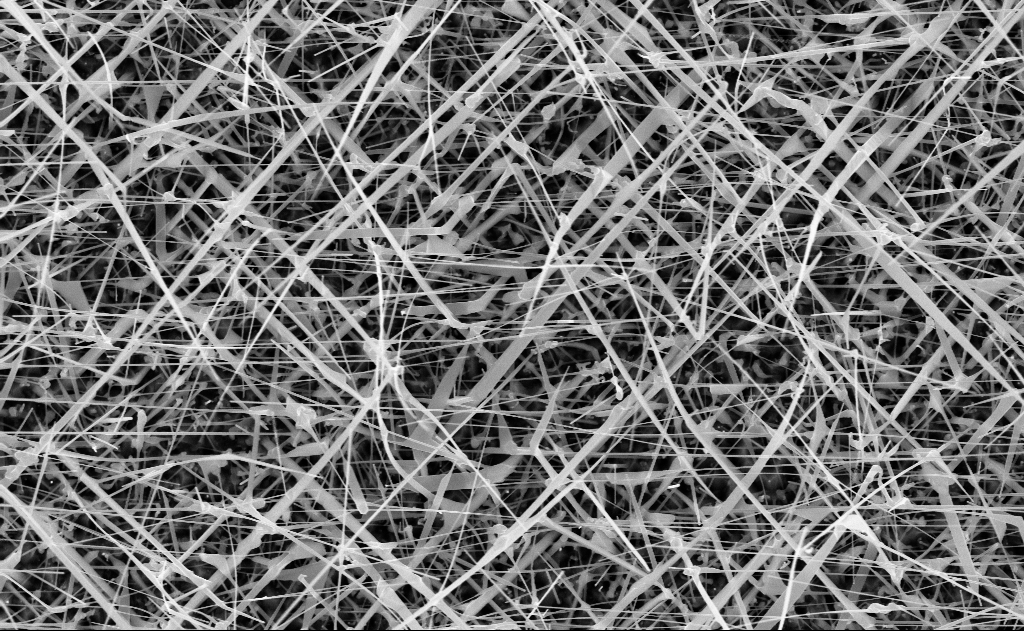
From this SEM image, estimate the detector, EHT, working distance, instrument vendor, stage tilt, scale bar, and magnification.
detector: InLens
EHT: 10 kV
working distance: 11 mm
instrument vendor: Zeiss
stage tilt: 0°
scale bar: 1000 nm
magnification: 20 K X